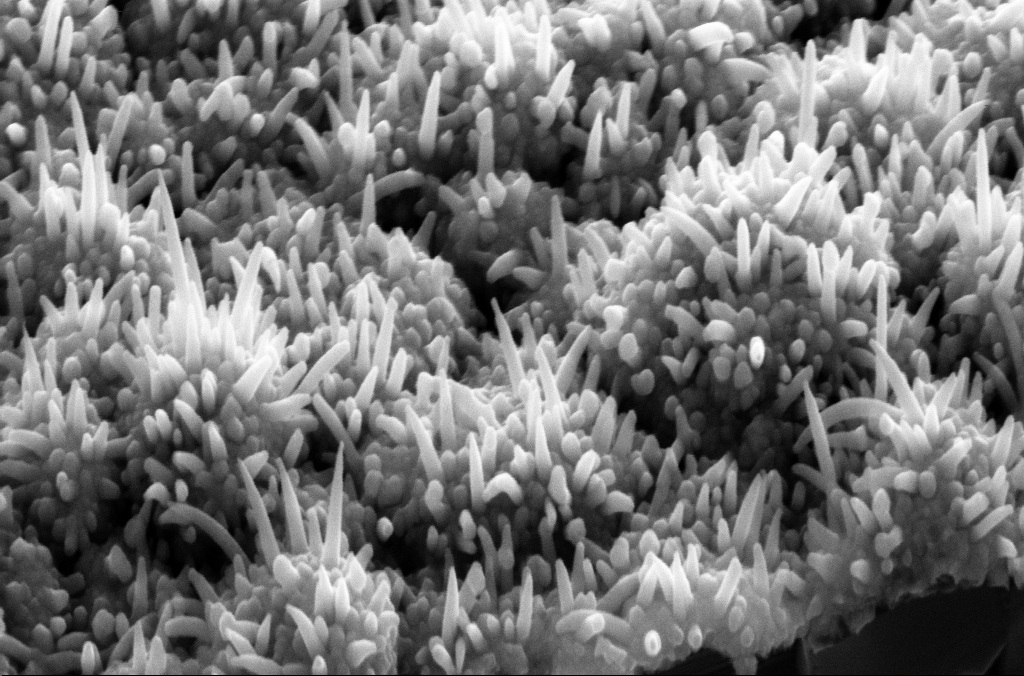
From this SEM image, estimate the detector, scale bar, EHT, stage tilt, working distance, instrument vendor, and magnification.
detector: SE2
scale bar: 200 nm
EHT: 10 kV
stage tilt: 45°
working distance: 8 mm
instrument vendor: Zeiss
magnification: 80.79 K X